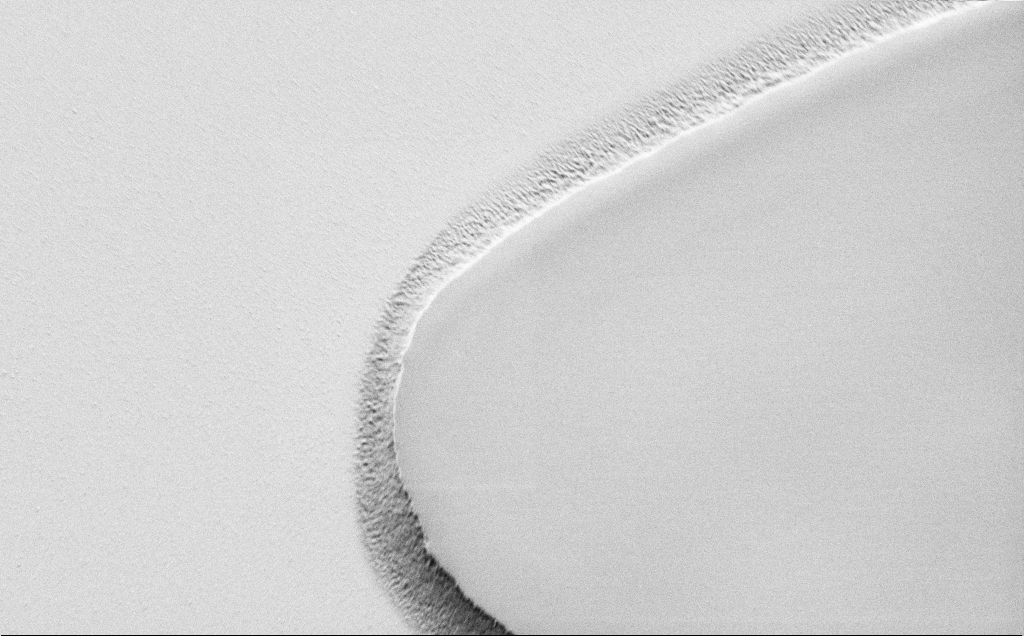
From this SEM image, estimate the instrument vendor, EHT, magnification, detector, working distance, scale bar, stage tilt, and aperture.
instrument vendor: Zeiss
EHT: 1.5 kV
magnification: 9.62 K X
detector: SE2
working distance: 7 mm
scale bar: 2000 nm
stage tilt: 45°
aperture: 30 µm